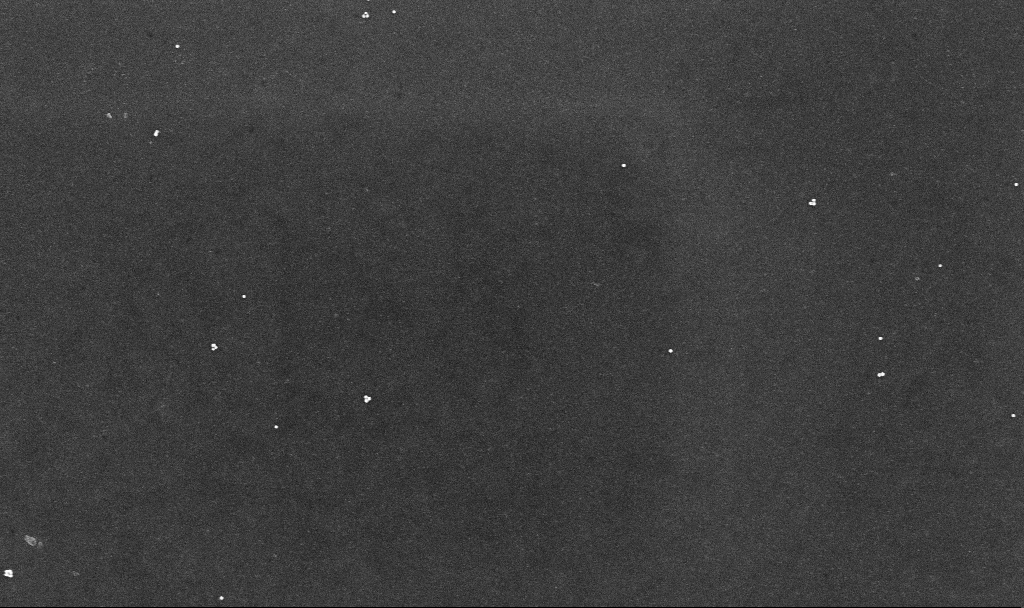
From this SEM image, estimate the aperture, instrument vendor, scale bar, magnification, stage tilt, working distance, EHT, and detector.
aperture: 30 µm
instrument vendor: Zeiss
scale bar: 1000 nm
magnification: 50 K X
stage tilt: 0°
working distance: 3.3 mm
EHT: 10 kV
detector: InLens